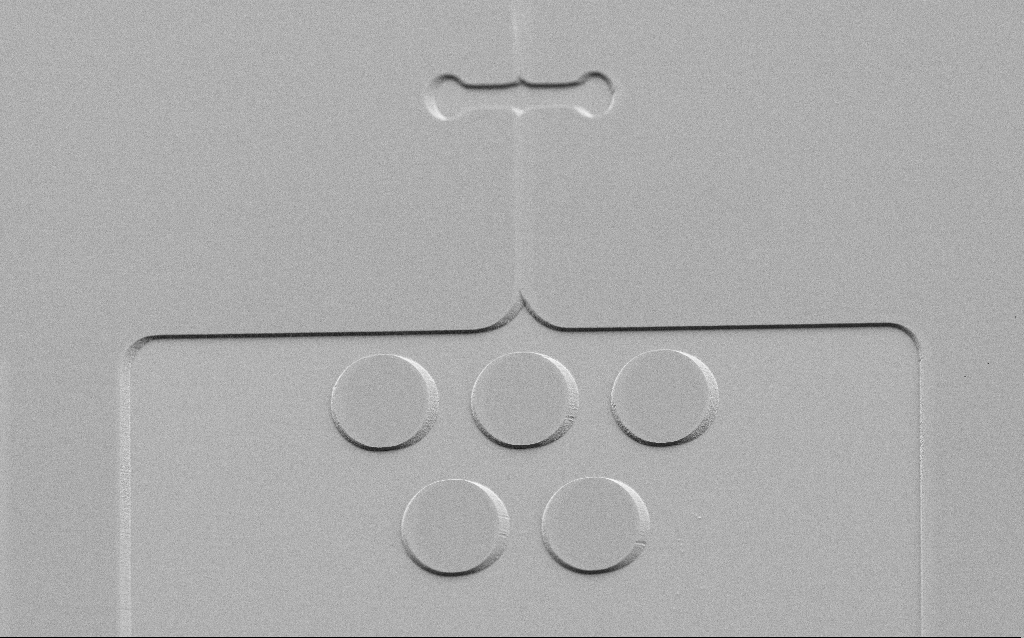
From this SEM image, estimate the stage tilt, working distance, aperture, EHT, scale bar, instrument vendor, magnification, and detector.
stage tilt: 45°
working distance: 6 mm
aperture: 30 µm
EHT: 3 kV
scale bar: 20000 nm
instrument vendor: Zeiss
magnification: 0.863 K X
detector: SE2